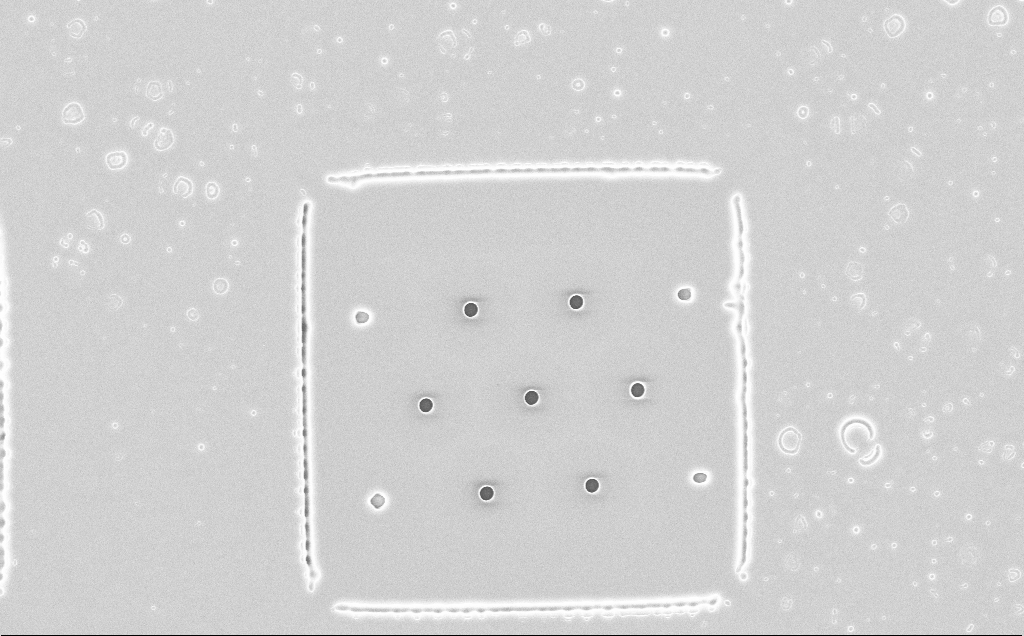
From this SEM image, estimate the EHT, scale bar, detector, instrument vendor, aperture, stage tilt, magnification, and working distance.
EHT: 10 kV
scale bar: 10000 nm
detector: InLens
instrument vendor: Zeiss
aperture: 30 µm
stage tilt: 0°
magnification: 1.93 K X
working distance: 13 mm